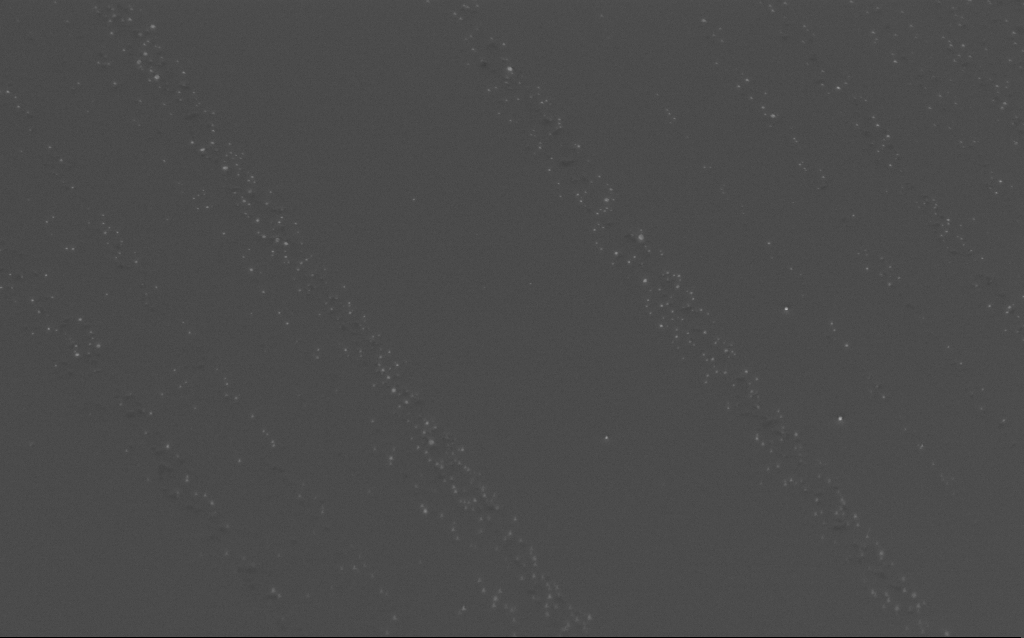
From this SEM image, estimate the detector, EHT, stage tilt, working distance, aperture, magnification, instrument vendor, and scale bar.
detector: InLens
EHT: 3 kV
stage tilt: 40°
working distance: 5 mm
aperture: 30 µm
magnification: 15.8 K X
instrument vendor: Zeiss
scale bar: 2000 nm